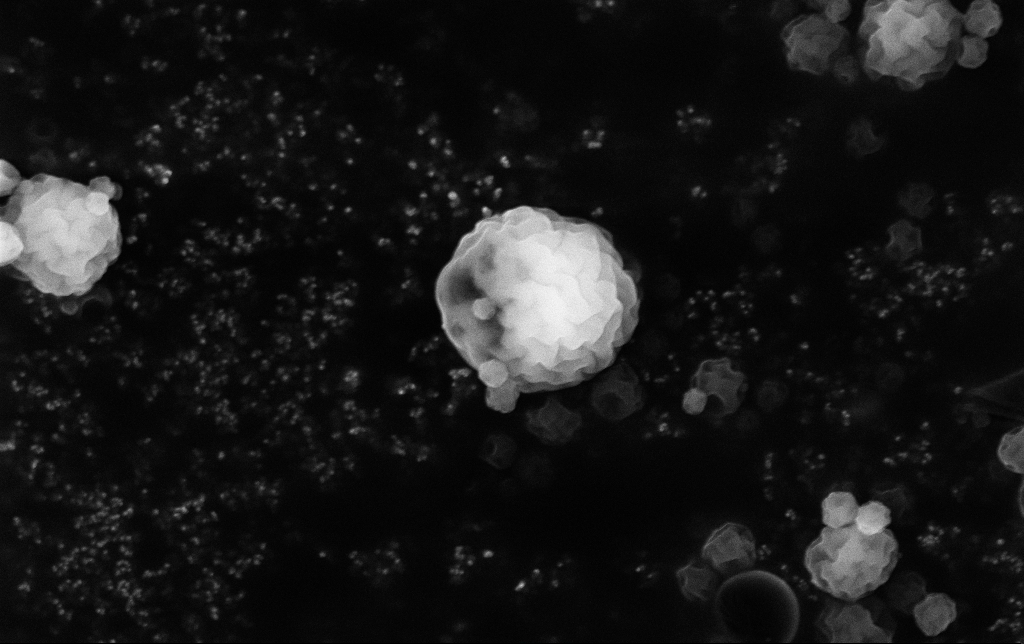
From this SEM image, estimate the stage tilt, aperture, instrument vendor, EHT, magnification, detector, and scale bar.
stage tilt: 0°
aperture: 30 µm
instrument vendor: Zeiss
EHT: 15 kV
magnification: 19.62 K X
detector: InLens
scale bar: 2000 nm